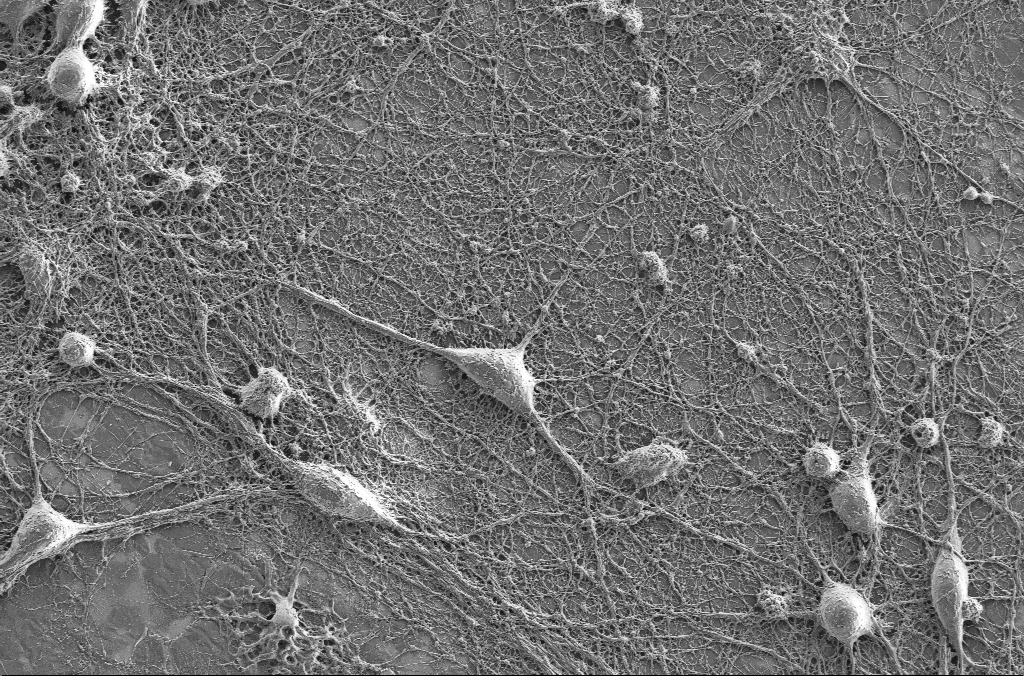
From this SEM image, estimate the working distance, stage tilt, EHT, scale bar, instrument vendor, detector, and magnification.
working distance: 4.1 mm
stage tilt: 0°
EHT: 2 kV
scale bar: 10000 nm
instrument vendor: Zeiss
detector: SE2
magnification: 2.5 K X